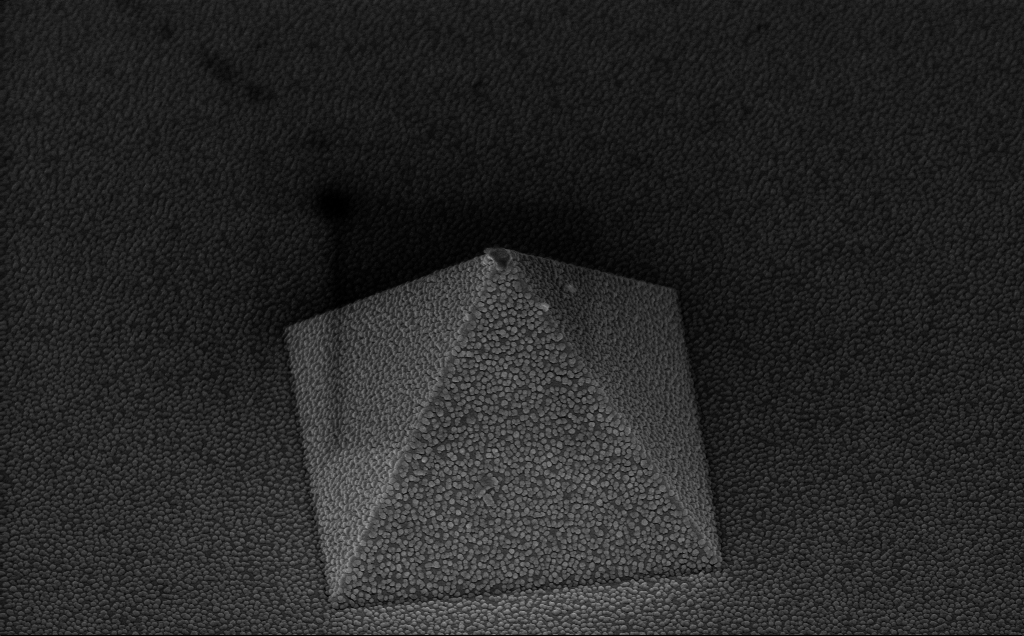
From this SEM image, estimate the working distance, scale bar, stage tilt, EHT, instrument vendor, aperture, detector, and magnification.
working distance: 8 mm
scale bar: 1000 nm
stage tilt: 45°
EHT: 10 kV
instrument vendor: Zeiss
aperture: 30 µm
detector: InLens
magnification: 28.82 K X